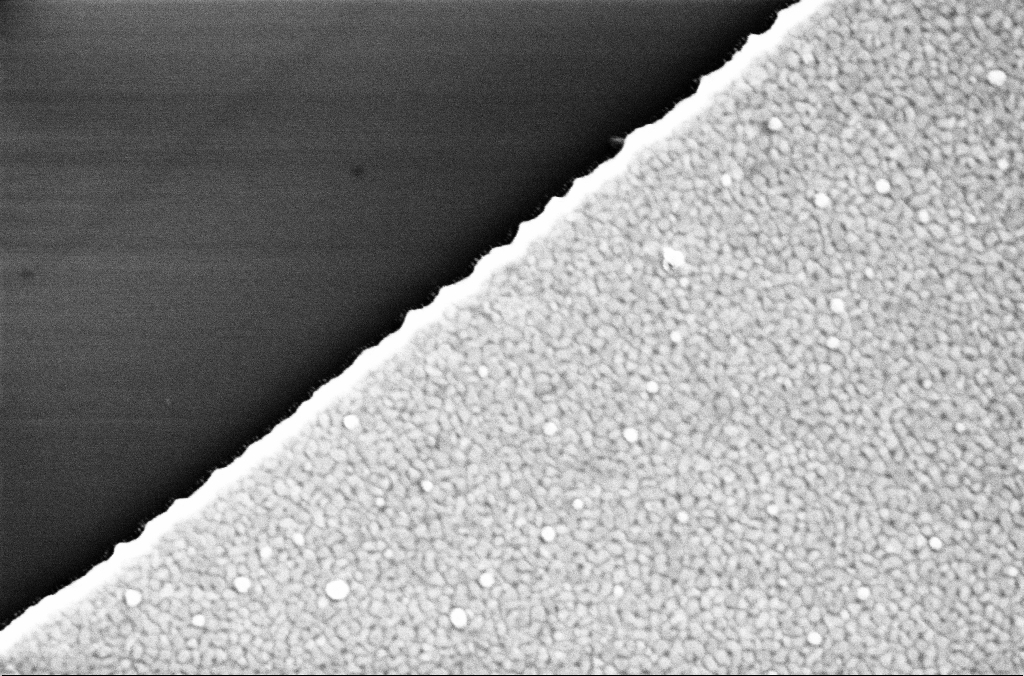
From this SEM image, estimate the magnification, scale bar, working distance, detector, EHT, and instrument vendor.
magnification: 94.3 K X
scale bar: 200 nm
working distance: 3.4 mm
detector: InLens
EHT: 5 kV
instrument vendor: Zeiss